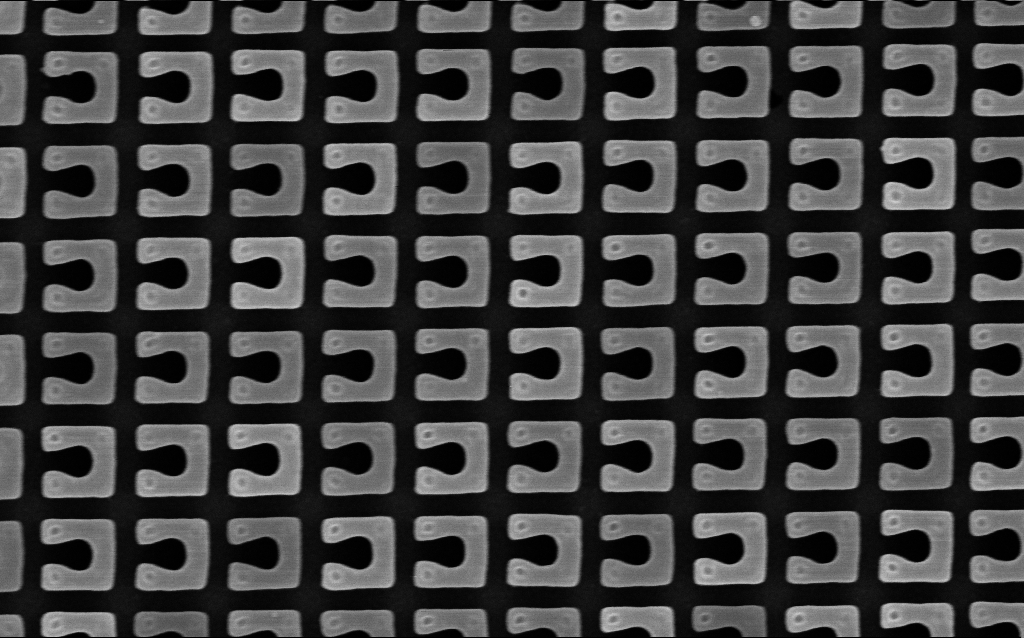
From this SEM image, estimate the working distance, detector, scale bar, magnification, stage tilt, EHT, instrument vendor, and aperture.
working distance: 4.3 mm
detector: InLens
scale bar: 200 nm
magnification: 74.34 K X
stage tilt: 0°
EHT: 3 kV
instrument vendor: Zeiss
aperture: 30 µm